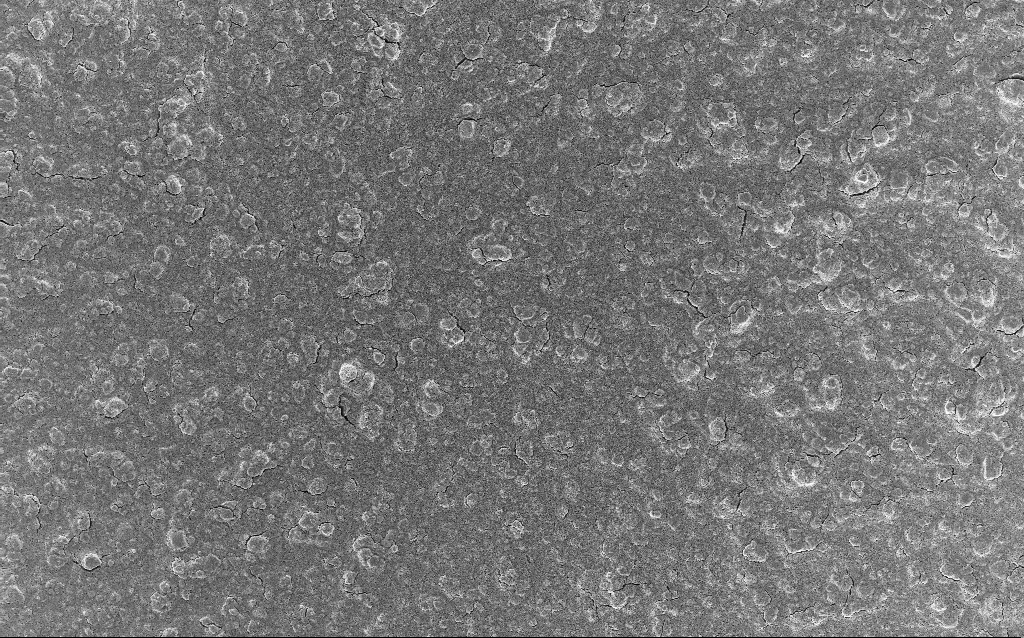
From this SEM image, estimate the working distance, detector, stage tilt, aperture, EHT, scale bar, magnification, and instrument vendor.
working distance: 3 mm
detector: InLens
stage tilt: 0°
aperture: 30 µm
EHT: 5 kV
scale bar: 20000 nm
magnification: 0.77 K X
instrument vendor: Zeiss